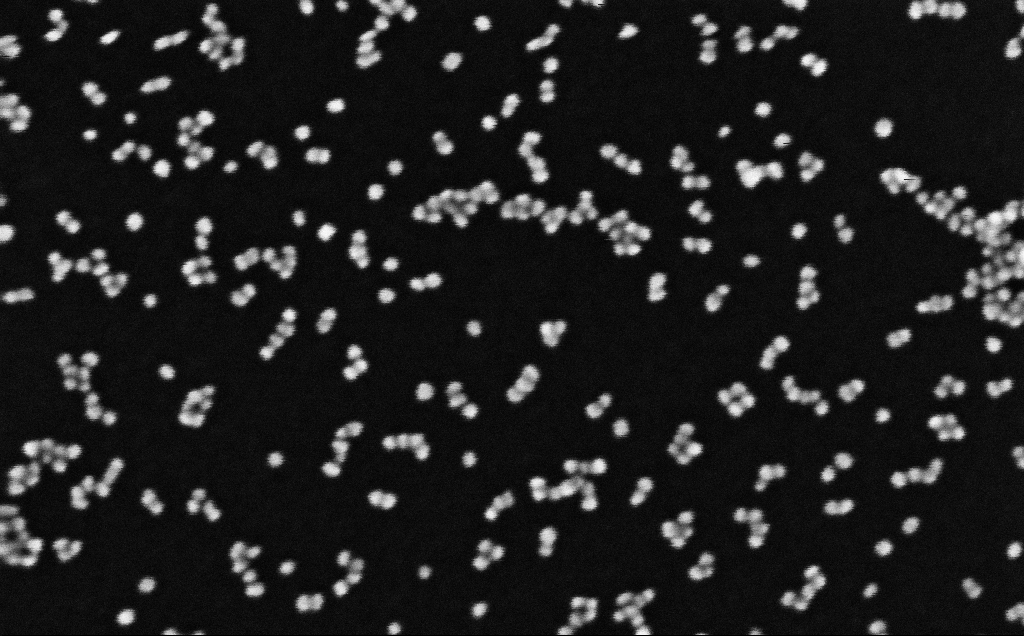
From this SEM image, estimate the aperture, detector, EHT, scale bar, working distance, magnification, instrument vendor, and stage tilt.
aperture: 30 µm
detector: InLens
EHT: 10 kV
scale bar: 100 nm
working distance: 4.1 mm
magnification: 622.7 K X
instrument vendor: Zeiss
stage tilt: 0°